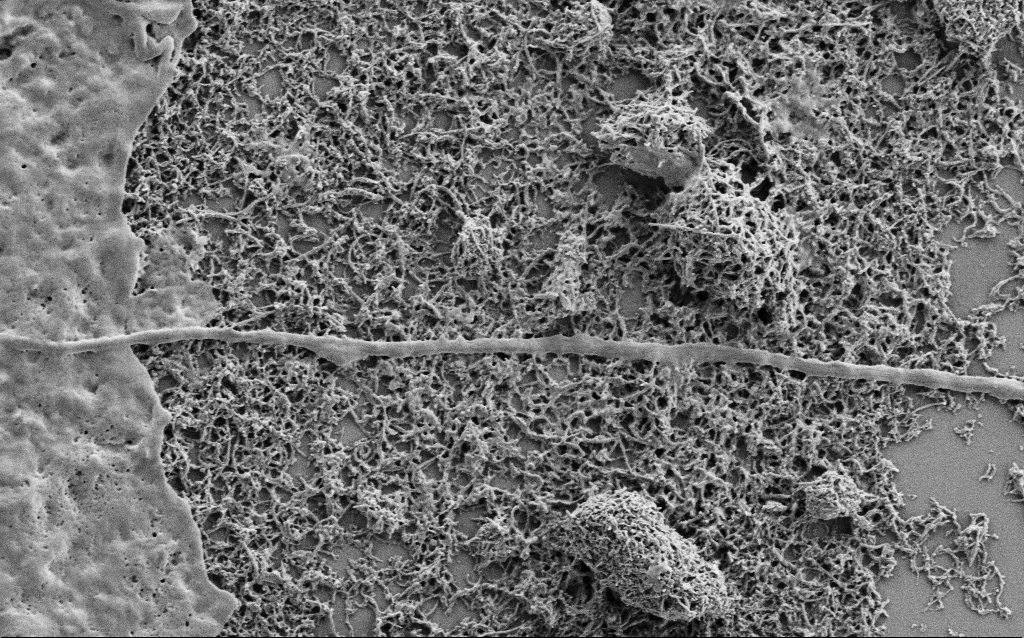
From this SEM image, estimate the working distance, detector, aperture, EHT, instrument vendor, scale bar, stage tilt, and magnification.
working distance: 7 mm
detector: SE2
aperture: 30 µm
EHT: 1 kV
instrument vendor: Zeiss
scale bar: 1000 nm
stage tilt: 0°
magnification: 25 K X